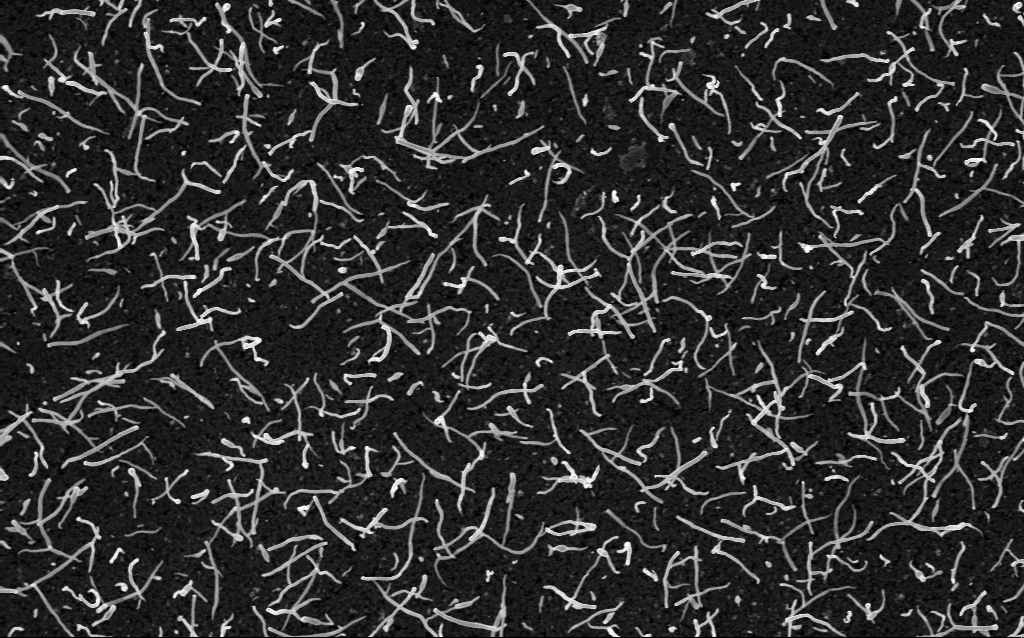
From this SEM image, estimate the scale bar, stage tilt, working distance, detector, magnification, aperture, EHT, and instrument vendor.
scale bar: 1000 nm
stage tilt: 0°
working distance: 1.8 mm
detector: InLens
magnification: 20 K X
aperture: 30 µm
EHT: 5 kV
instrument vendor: Zeiss